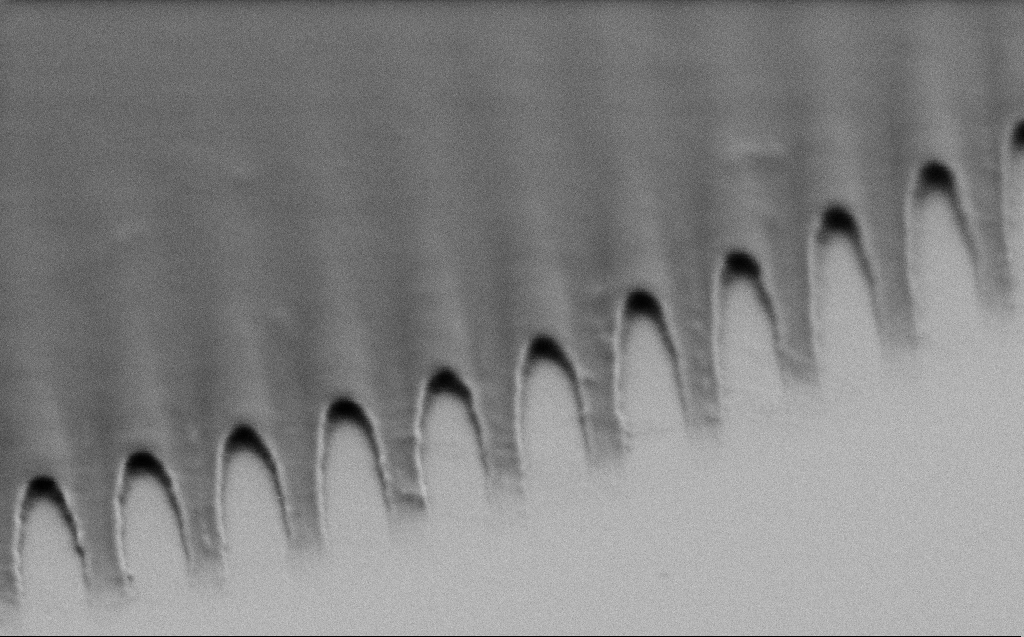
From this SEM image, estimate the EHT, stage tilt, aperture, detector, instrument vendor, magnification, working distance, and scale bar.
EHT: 1 kV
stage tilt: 60.7°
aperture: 30 µm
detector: SE2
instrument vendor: Zeiss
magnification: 76.56 K X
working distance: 3 mm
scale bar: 200 nm